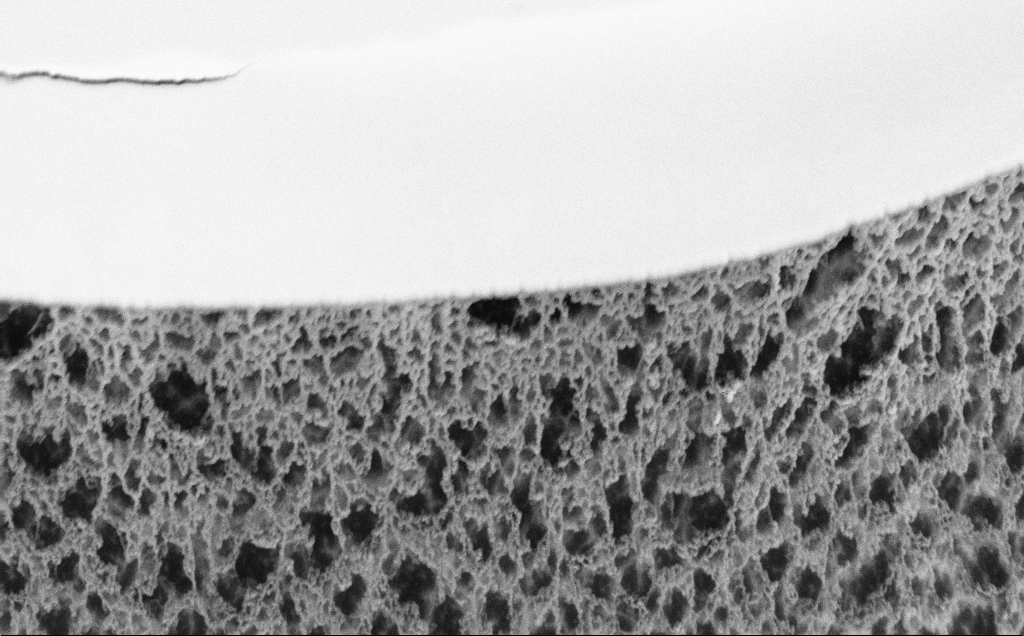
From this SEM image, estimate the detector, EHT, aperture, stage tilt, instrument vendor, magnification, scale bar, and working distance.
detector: SE2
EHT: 5 kV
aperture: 30 µm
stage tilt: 45°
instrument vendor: Zeiss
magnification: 20.23 K X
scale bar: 2000 nm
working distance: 8 mm